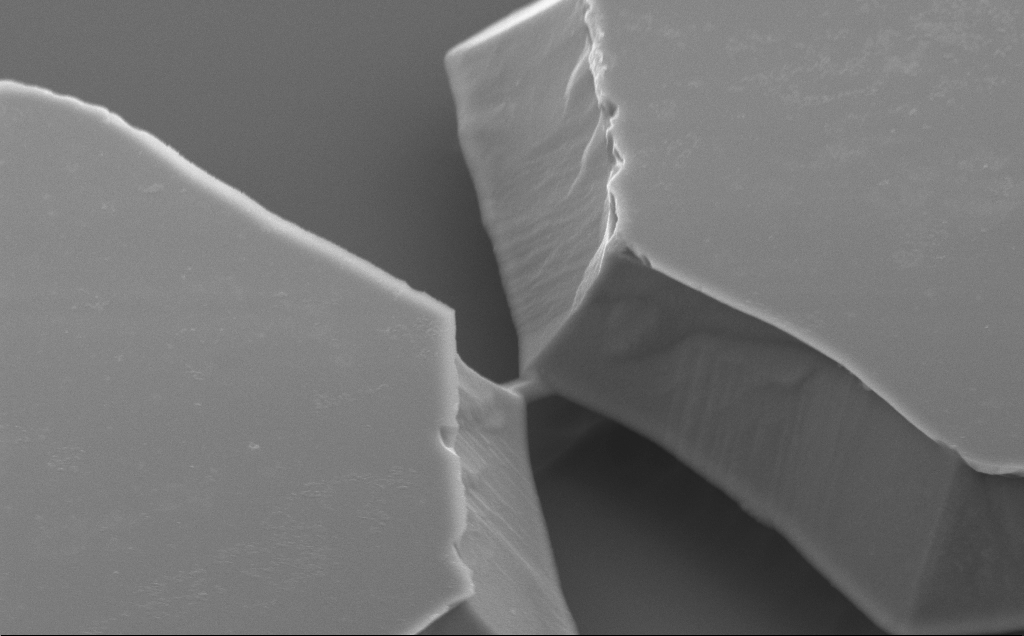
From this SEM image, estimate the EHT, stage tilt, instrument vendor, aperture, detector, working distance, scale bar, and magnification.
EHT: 5 kV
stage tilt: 50°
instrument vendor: Zeiss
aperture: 30 µm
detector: SE2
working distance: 10 mm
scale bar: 2000 nm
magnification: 21.92 K X